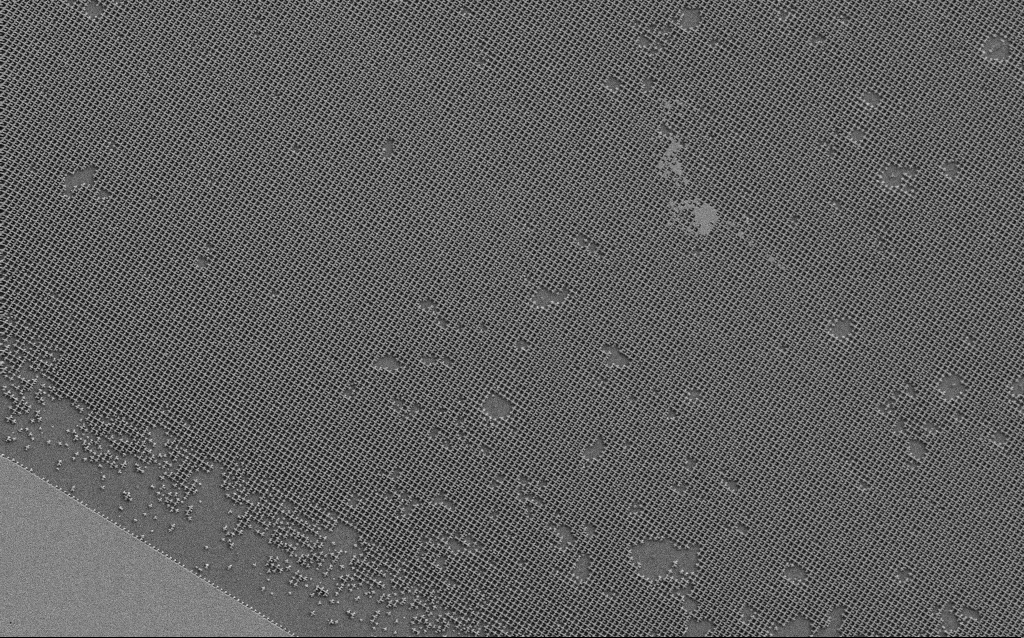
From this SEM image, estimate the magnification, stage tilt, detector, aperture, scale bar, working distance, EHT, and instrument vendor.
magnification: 4.01 K X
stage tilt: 0°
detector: SE2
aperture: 30 µm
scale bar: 10000 nm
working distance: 6.1 mm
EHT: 5 kV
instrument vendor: Zeiss